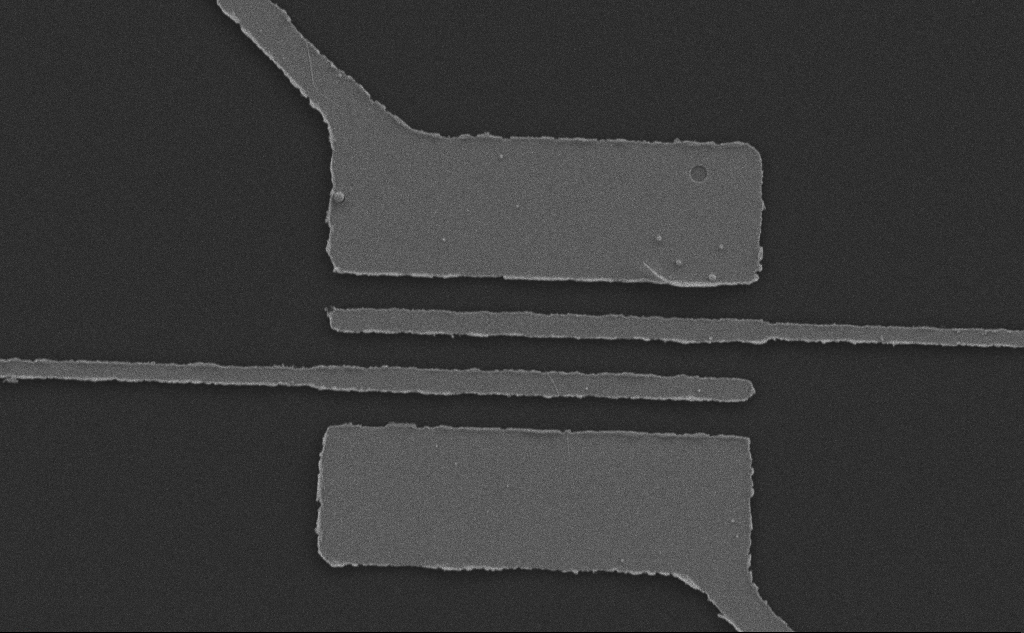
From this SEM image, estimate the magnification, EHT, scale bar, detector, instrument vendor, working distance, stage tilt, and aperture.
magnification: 5.46 K X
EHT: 10 kV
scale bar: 10000 nm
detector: SE2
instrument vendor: Zeiss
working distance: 6 mm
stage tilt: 0°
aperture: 30 µm